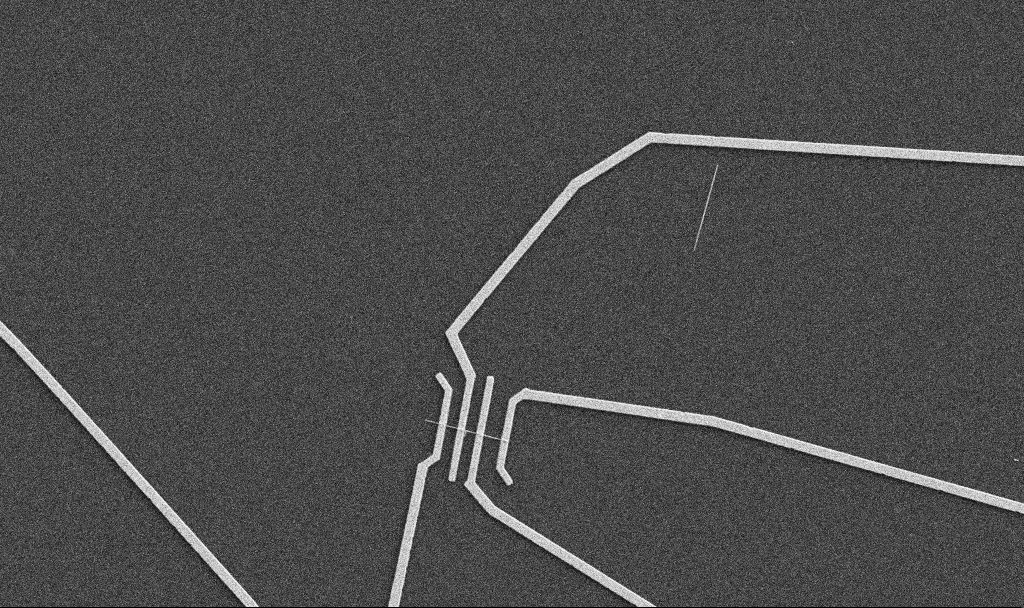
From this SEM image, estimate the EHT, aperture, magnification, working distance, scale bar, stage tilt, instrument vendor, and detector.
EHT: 5 kV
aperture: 30 µm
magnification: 5 K X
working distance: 10.7 mm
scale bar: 10000 nm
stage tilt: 0°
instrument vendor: Zeiss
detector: SE2